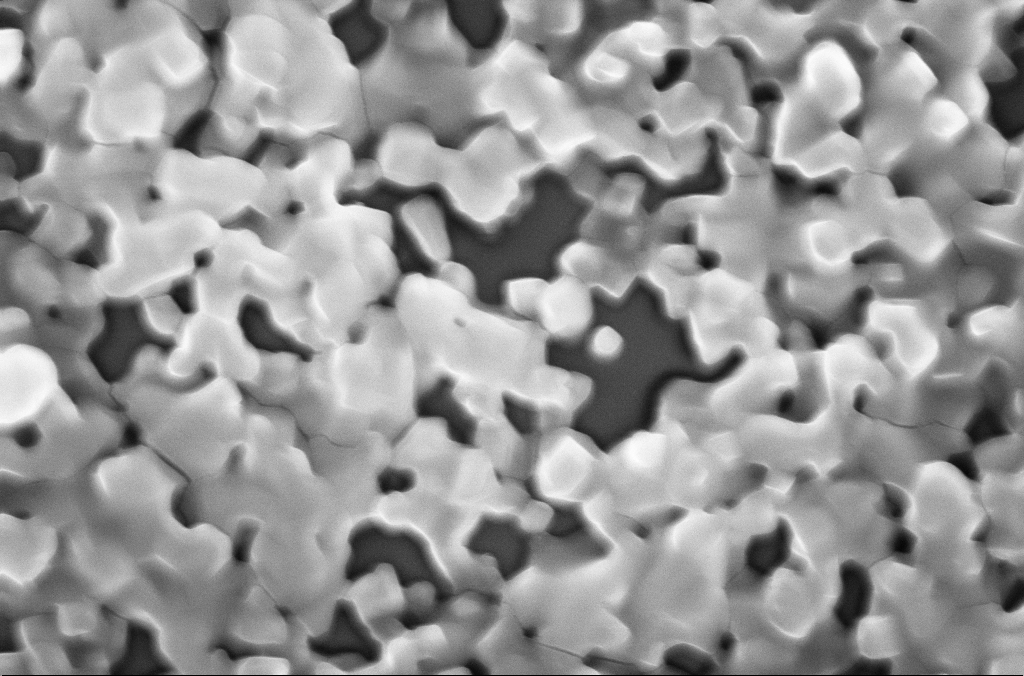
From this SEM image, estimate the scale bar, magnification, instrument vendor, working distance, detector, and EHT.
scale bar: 1000 nm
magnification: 50 K X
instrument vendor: Zeiss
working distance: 2.7 mm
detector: InLens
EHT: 20 kV